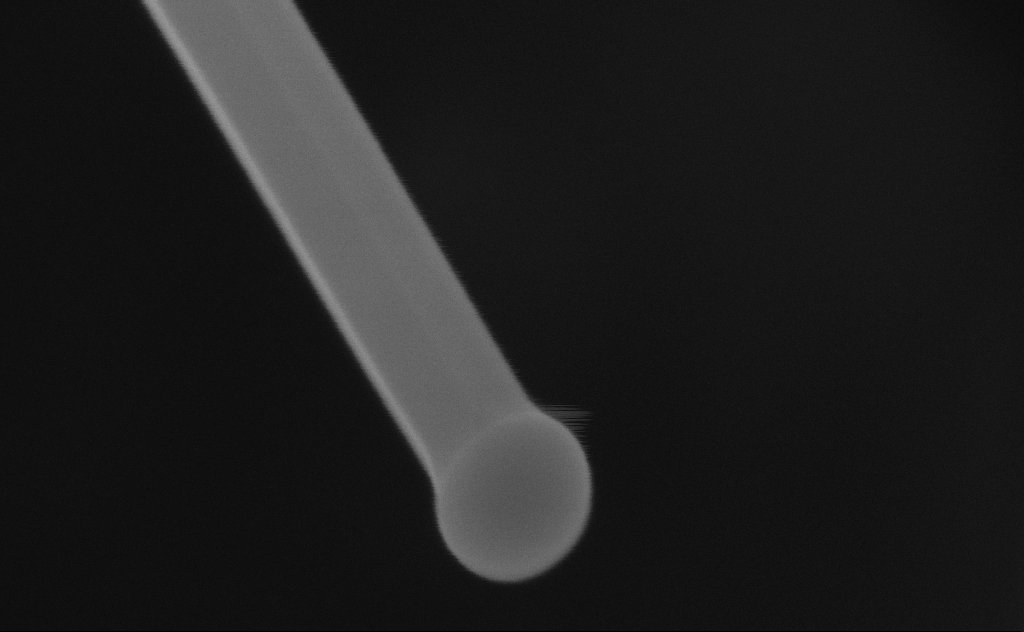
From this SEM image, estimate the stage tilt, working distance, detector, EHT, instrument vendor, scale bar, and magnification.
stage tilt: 0°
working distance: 6 mm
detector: InLens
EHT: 10 kV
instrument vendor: Zeiss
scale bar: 100 nm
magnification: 427.08 K X